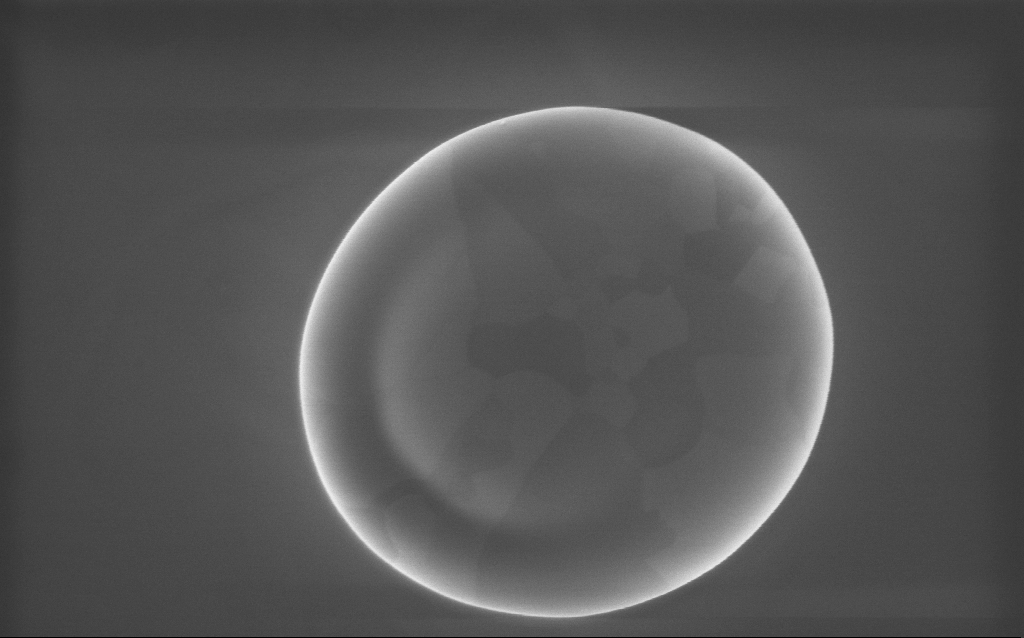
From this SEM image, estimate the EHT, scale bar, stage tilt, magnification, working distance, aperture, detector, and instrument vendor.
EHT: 10 kV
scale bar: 1000 nm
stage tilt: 0°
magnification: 58 K X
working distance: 2 mm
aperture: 30 µm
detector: InLens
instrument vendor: Zeiss